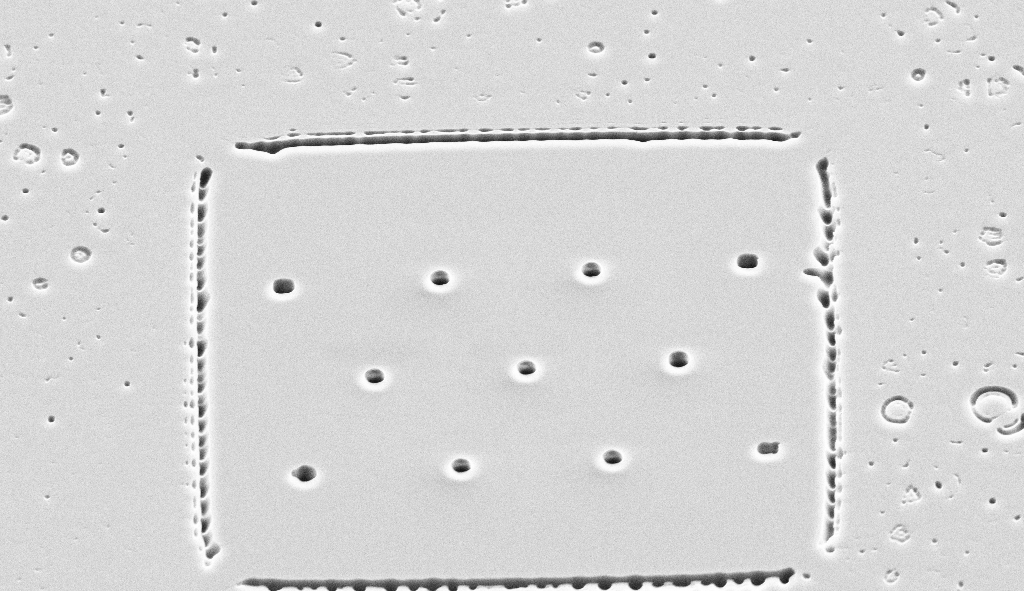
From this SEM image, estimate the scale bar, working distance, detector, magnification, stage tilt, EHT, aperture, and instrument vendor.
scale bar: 20000 nm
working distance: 13 mm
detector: SE2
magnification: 2.76 K X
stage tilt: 45°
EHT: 10 kV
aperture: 30 µm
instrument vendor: Zeiss